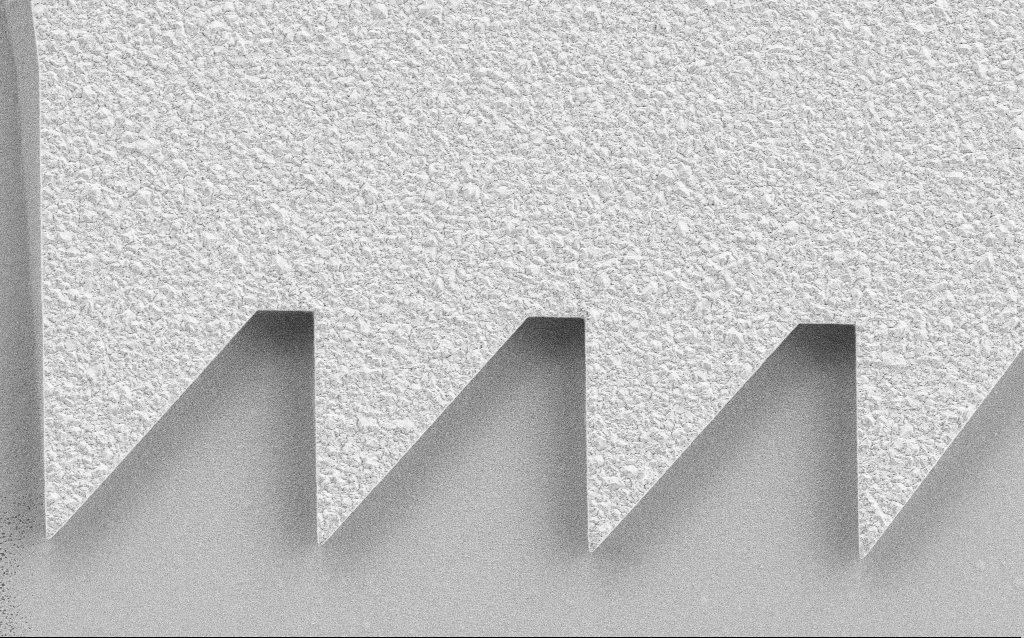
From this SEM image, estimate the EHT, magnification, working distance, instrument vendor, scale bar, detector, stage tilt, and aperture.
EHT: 3 kV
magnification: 8.79 K X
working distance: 4.4 mm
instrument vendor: Zeiss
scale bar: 2000 nm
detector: SE2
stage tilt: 0°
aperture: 30 µm